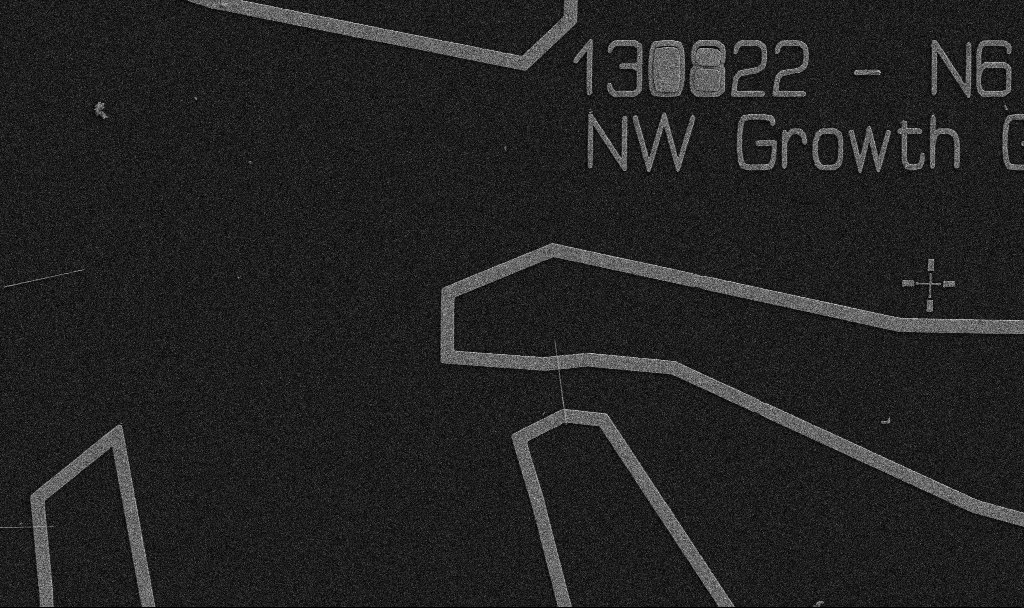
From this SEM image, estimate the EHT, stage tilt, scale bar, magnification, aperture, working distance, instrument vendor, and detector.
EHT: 5 kV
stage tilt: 0°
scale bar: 10000 nm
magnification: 5 K X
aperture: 30 µm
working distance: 10.7 mm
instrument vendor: Zeiss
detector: SE2